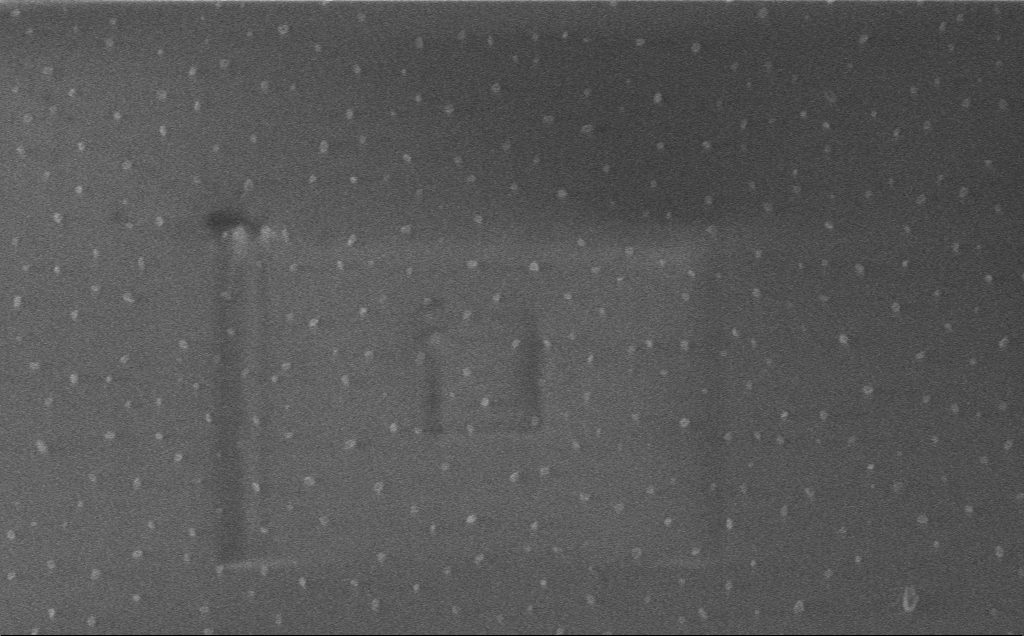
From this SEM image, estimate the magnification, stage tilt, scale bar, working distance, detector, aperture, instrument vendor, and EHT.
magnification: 42.85 K X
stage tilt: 0°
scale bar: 1000 nm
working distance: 3 mm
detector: InLens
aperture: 30 µm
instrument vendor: Zeiss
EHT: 1 kV